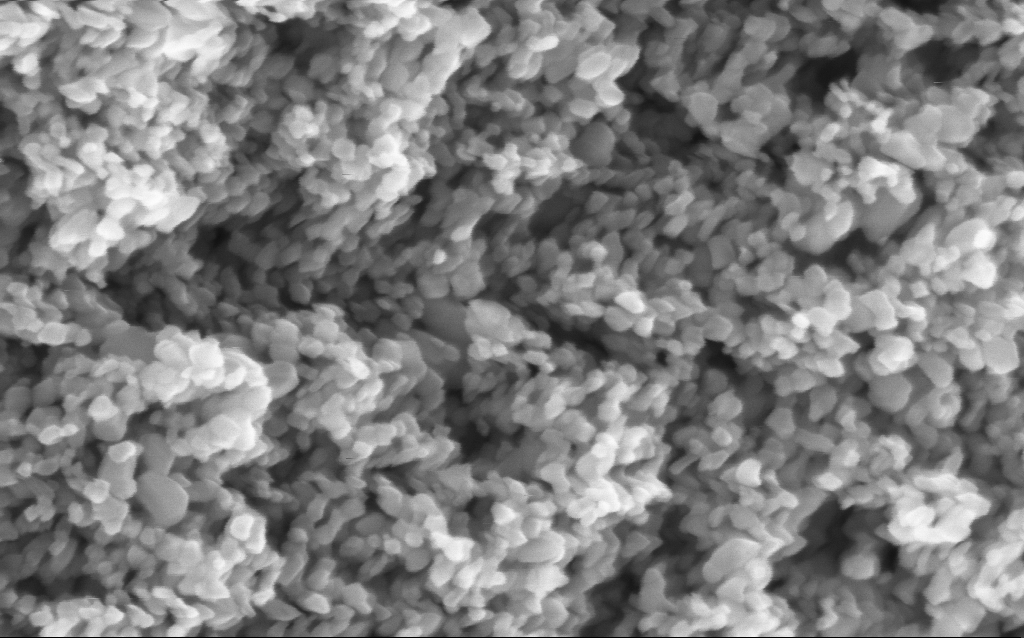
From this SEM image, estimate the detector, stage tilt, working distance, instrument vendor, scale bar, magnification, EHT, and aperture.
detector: InLens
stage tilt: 0°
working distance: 4.5 mm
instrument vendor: Zeiss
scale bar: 100 nm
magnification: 294.27 K X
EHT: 5 kV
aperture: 30 µm